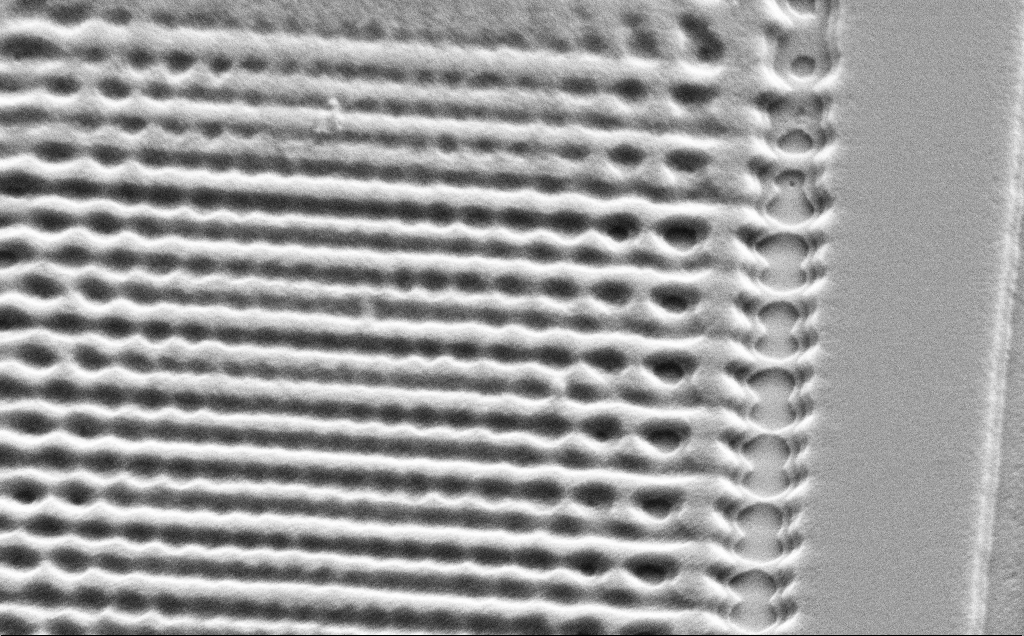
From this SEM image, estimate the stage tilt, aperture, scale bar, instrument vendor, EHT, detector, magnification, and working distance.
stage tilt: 45°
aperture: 30 µm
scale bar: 2000 nm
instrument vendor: Zeiss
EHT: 5 kV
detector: SE2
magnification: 12.64 K X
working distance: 9 mm